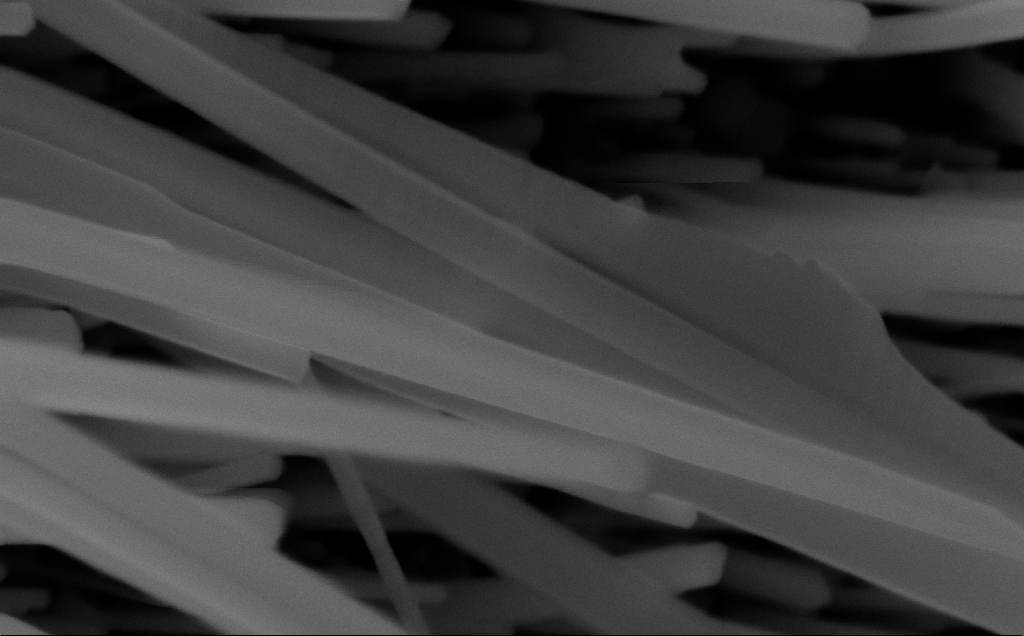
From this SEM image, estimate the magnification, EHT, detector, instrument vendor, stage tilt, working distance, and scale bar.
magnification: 159.62 K X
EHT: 10 kV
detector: InLens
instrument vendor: Zeiss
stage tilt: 0°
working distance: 6 mm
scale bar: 200 nm